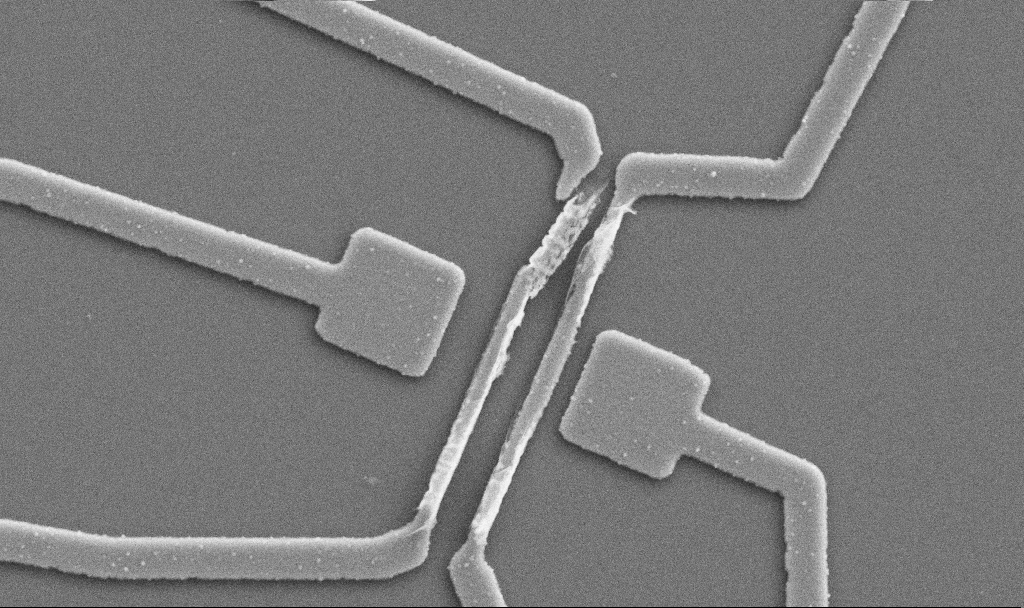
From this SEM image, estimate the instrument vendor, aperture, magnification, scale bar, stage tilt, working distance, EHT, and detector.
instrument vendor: Zeiss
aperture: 30 µm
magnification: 20 K X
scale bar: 1000 nm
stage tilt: -0°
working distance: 10.7 mm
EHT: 5 kV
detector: SE2